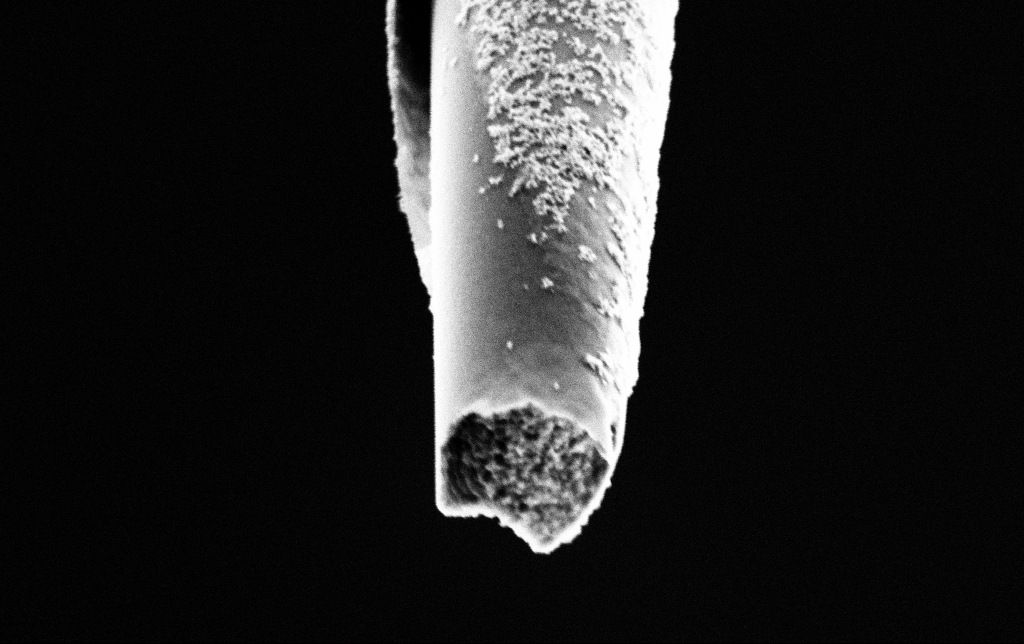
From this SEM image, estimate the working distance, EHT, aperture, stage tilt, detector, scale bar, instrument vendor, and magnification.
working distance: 7.3 mm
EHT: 2 kV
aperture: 30 µm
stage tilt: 45°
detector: SE2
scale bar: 1000 nm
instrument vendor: Zeiss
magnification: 50 K X